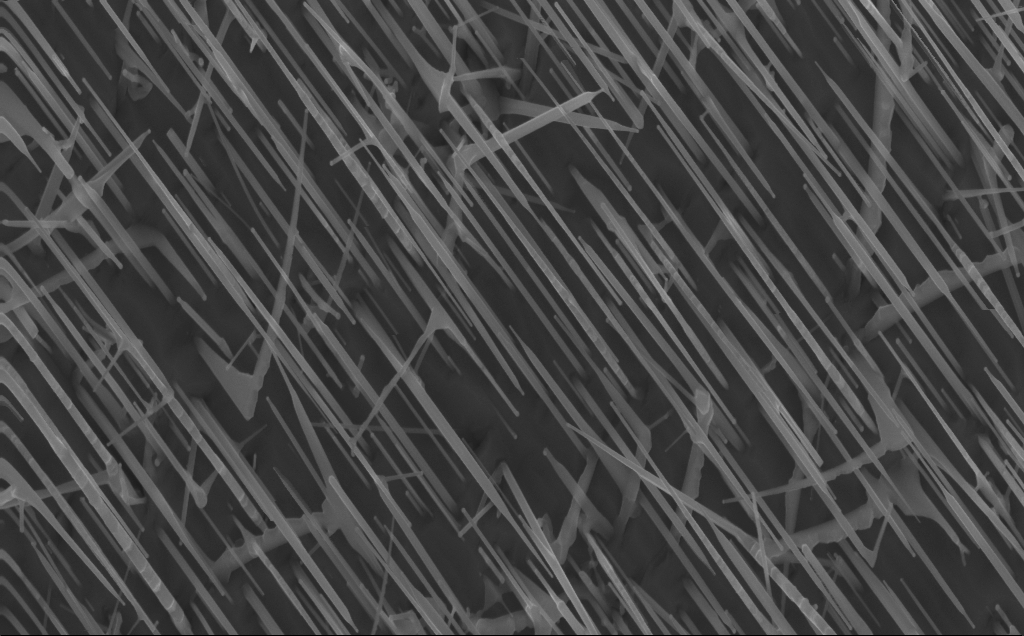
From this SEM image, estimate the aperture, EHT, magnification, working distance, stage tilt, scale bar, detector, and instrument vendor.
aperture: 30 µm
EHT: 10 kV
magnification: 40 K X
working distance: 4 mm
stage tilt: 0°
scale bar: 1000 nm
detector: InLens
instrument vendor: Zeiss